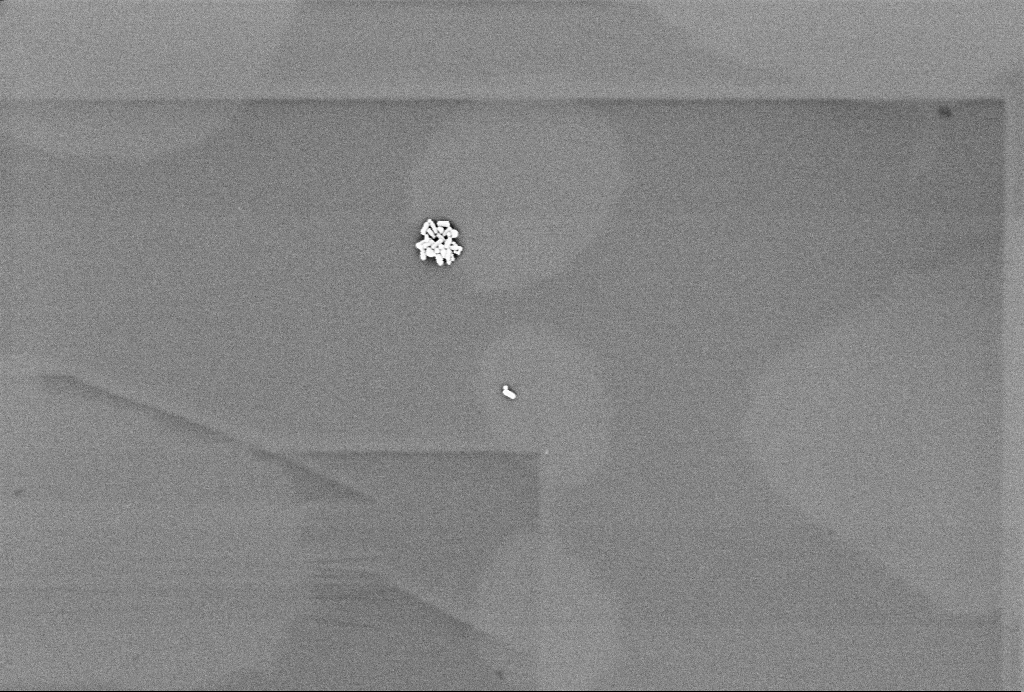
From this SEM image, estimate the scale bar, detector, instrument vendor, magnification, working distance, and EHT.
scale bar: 200 nm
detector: InLens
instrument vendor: Zeiss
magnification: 65 K X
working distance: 3.3 mm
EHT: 1 kV